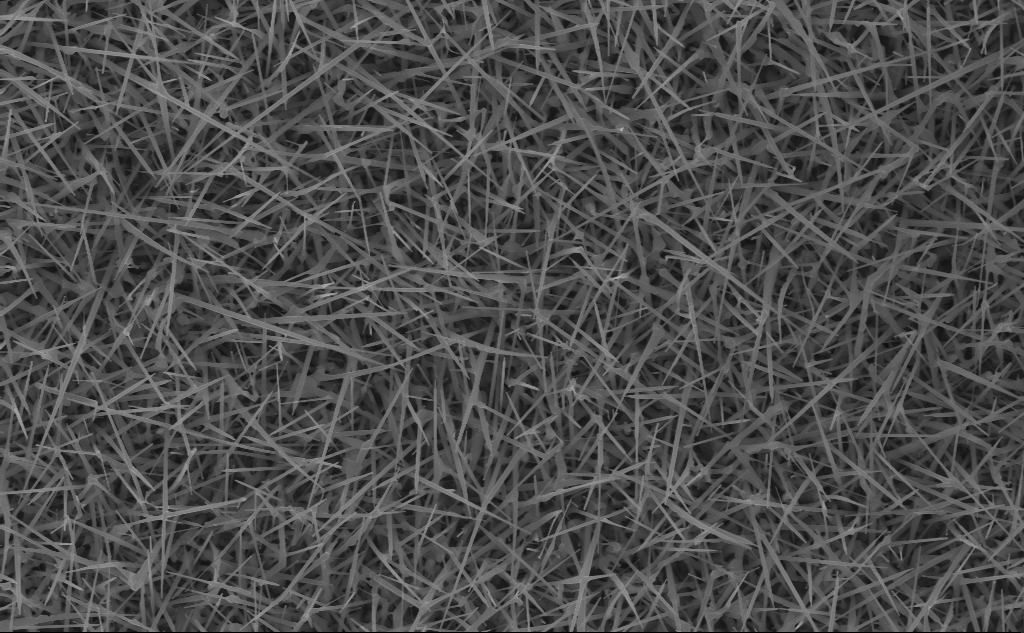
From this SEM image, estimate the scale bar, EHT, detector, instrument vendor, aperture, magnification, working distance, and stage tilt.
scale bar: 1000 nm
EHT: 10 kV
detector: InLens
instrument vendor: Zeiss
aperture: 30 µm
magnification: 20 K X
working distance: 7 mm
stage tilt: -0°